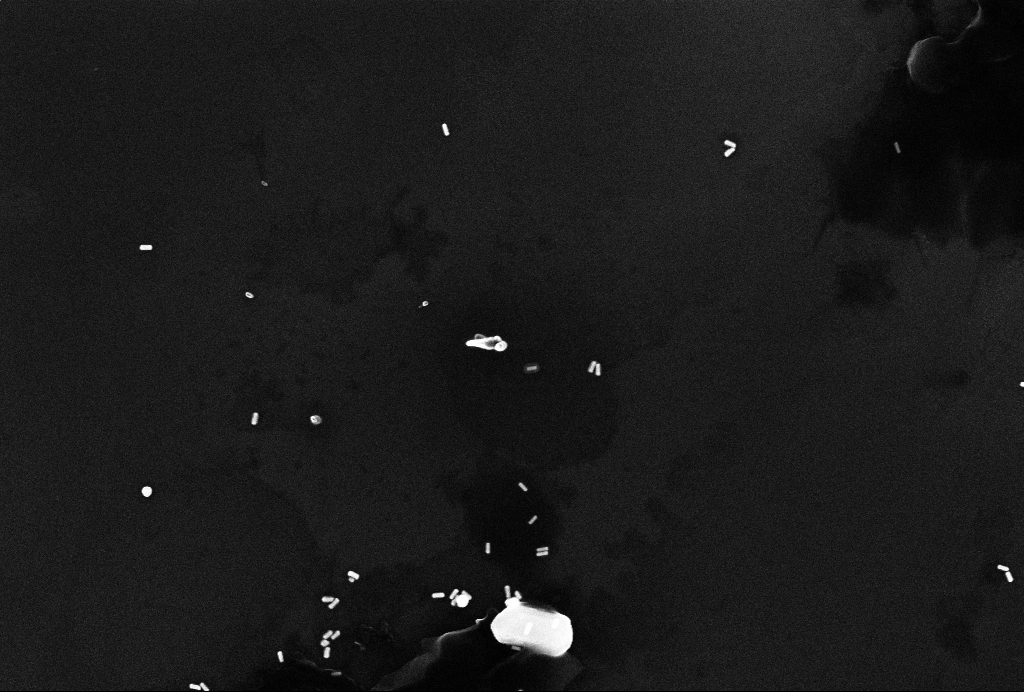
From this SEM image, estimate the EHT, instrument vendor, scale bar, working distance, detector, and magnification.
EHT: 10 kV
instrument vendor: Zeiss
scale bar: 1000 nm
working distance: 3.2 mm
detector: InLens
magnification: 60 K X